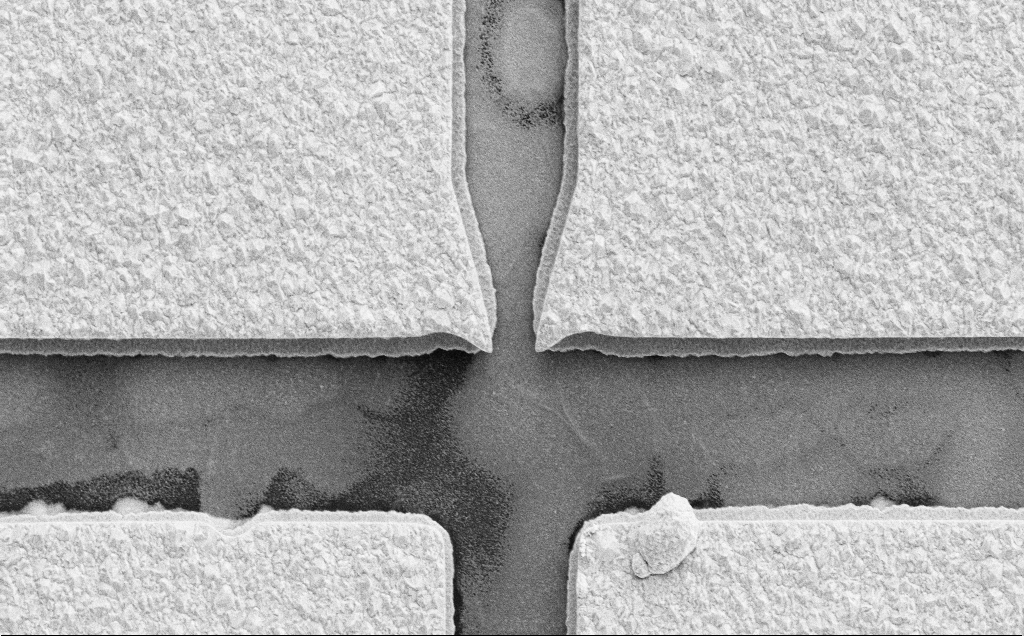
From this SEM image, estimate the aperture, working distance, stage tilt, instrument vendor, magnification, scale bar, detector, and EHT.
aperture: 30 µm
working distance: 14 mm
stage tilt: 0°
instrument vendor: Zeiss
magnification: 4.89 K X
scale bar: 10000 nm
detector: SE2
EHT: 10 kV